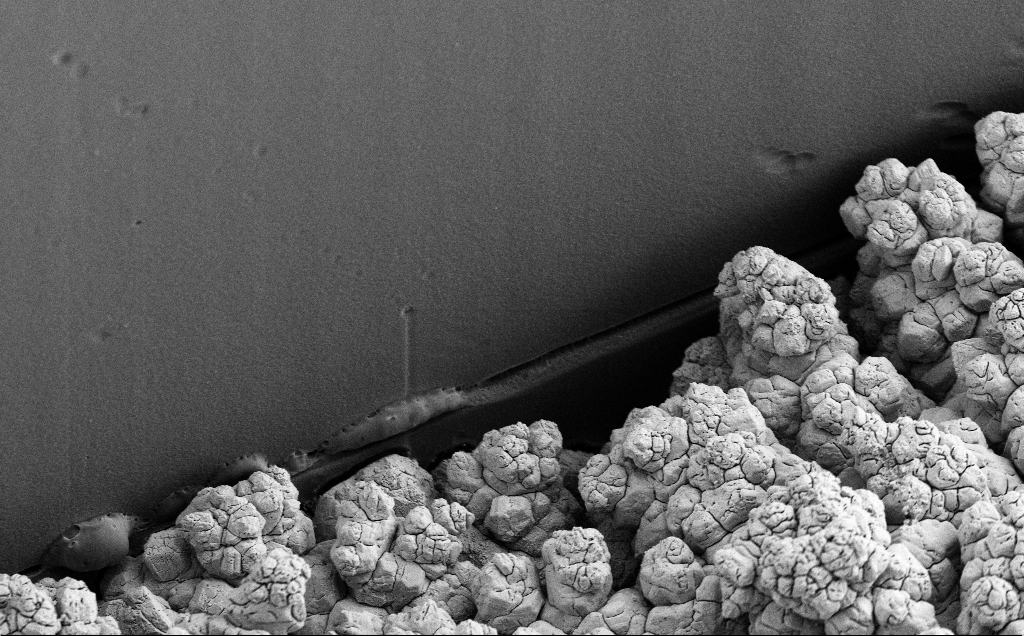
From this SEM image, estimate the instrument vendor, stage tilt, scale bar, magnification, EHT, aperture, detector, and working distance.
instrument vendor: Zeiss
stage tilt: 35°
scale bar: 20000 nm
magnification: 3.02 K X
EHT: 5 kV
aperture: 30 µm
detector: SE2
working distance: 9 mm